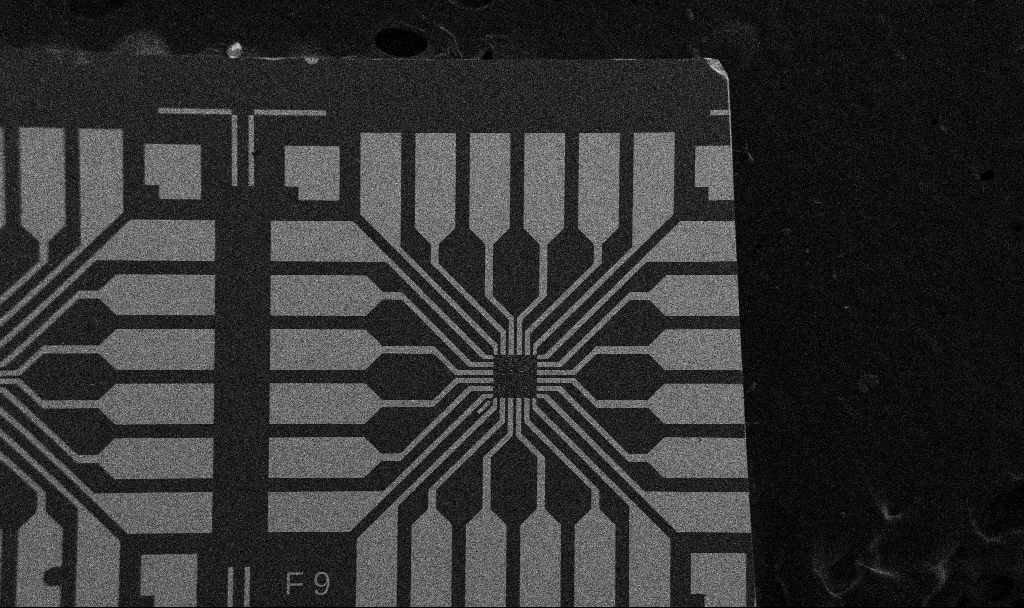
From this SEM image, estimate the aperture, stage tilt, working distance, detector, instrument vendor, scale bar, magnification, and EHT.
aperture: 30 µm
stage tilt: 0°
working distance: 10.7 mm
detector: SE2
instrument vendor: Zeiss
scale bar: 200000 nm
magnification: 0.1 K X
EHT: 5 kV